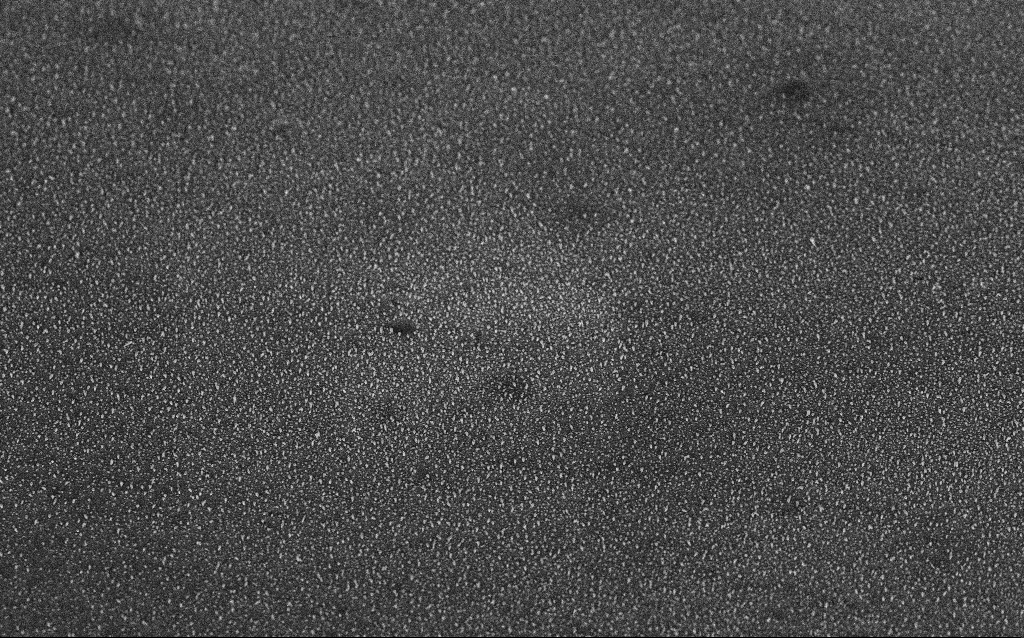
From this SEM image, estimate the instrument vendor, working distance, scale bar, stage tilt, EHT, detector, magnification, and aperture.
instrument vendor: Zeiss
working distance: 6.2 mm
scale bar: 2000 nm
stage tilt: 45°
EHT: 5 kV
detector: InLens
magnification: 10 K X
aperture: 30 µm